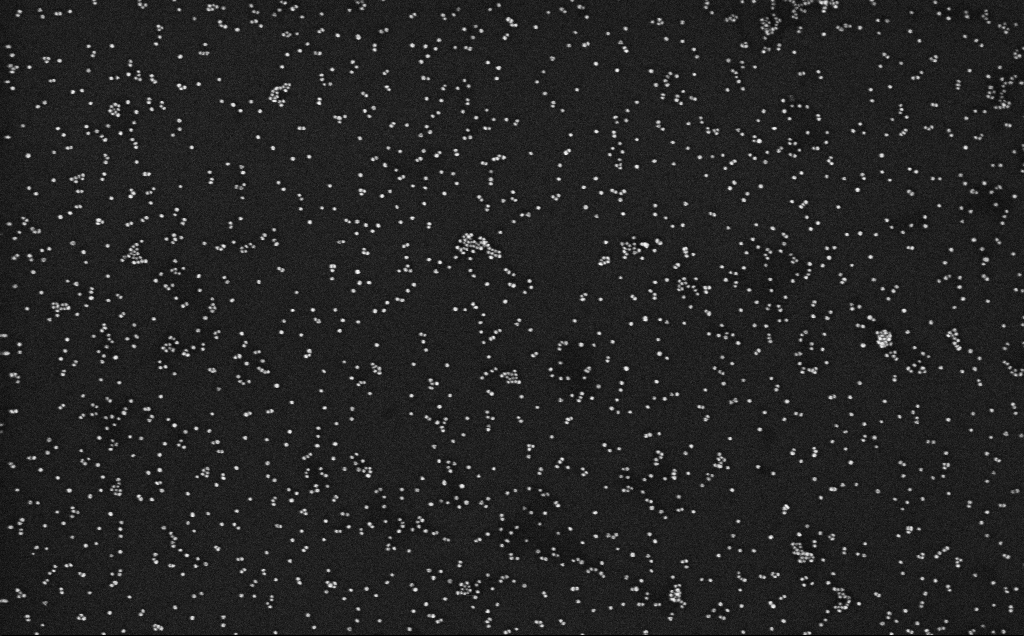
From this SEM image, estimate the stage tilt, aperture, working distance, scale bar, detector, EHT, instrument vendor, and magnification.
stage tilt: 0°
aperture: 30 µm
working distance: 3.2 mm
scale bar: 200 nm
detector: InLens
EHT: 10 kV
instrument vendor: Zeiss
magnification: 100 K X